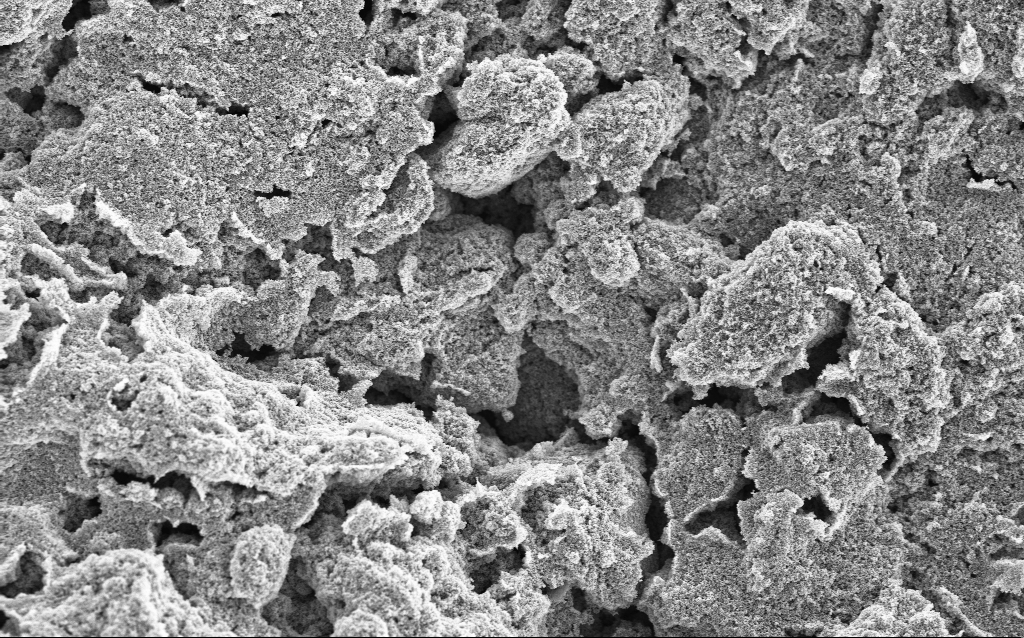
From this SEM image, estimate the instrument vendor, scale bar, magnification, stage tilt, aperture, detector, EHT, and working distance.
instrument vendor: Zeiss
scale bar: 10000 nm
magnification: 6.42 K X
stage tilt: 0°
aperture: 30 µm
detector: InLens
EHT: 5 kV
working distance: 4.4 mm